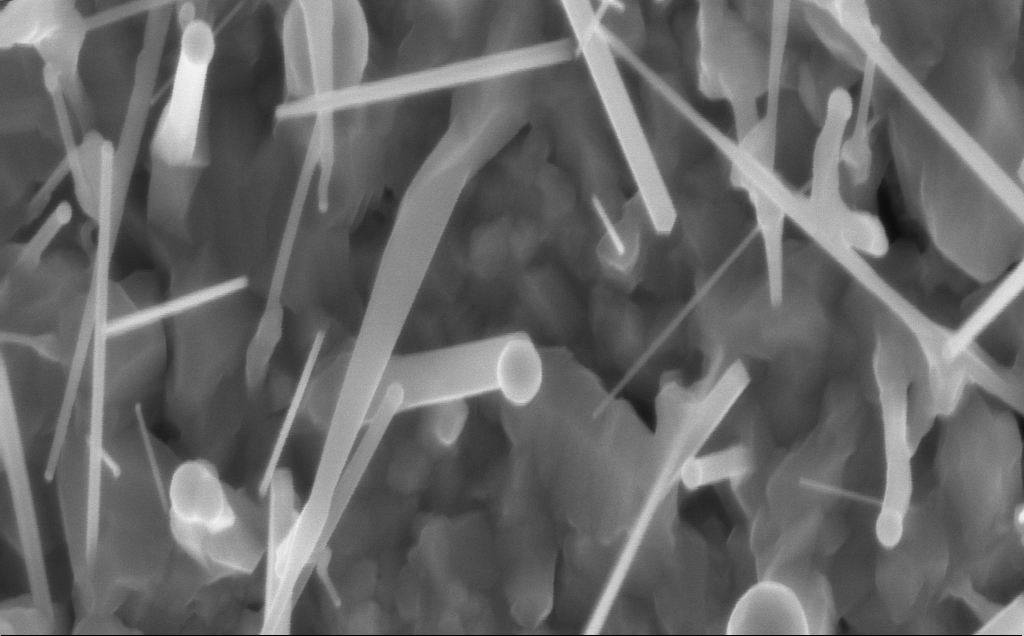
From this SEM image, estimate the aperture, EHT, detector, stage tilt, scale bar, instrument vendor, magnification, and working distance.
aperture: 30 µm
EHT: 10 kV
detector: InLens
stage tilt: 0°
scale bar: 1000 nm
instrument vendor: Zeiss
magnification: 70.88 K X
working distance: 4 mm